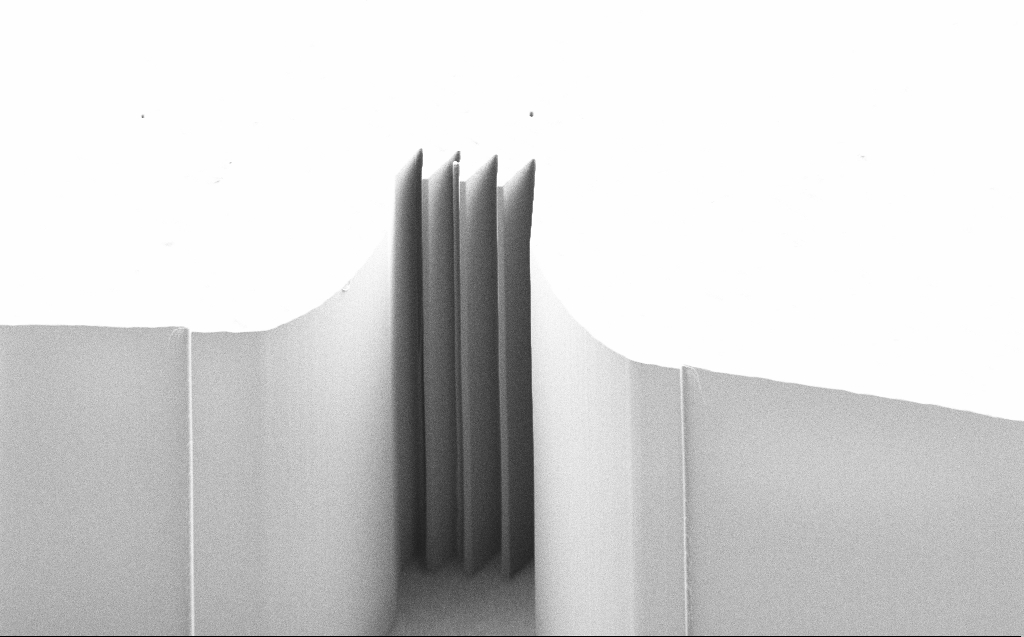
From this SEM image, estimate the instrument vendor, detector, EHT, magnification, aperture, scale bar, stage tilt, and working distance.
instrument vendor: Zeiss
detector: SE2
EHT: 1 kV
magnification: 1.21 K X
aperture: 30 µm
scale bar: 10000 nm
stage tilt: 44.9°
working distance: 7 mm